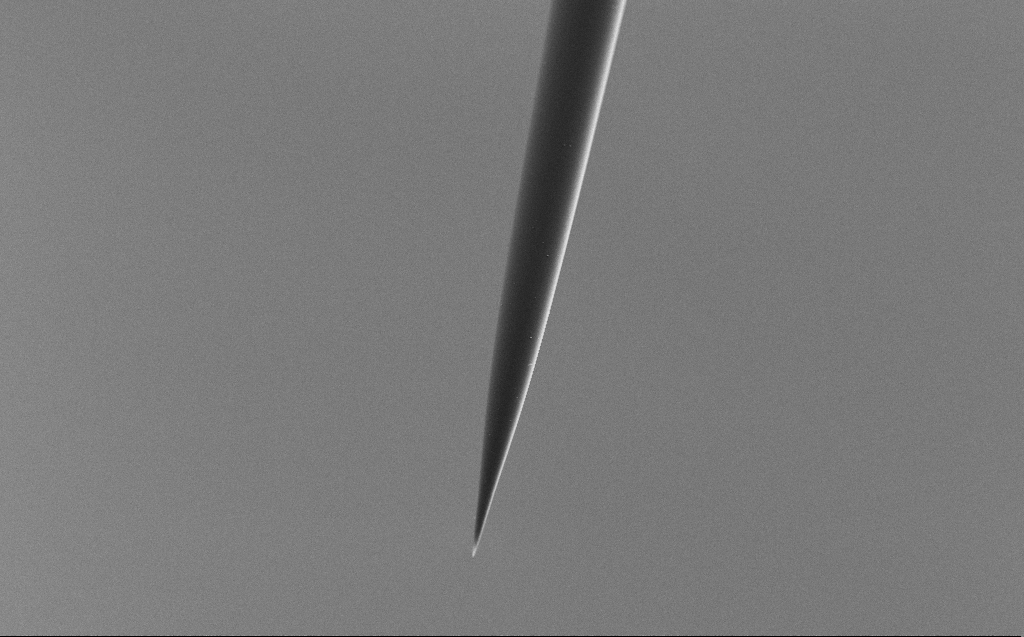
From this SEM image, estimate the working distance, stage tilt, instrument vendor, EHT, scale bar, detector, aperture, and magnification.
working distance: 5 mm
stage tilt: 45.1°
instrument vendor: Zeiss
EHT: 5 kV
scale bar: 20000 nm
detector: SE2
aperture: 30 µm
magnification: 1.51 K X